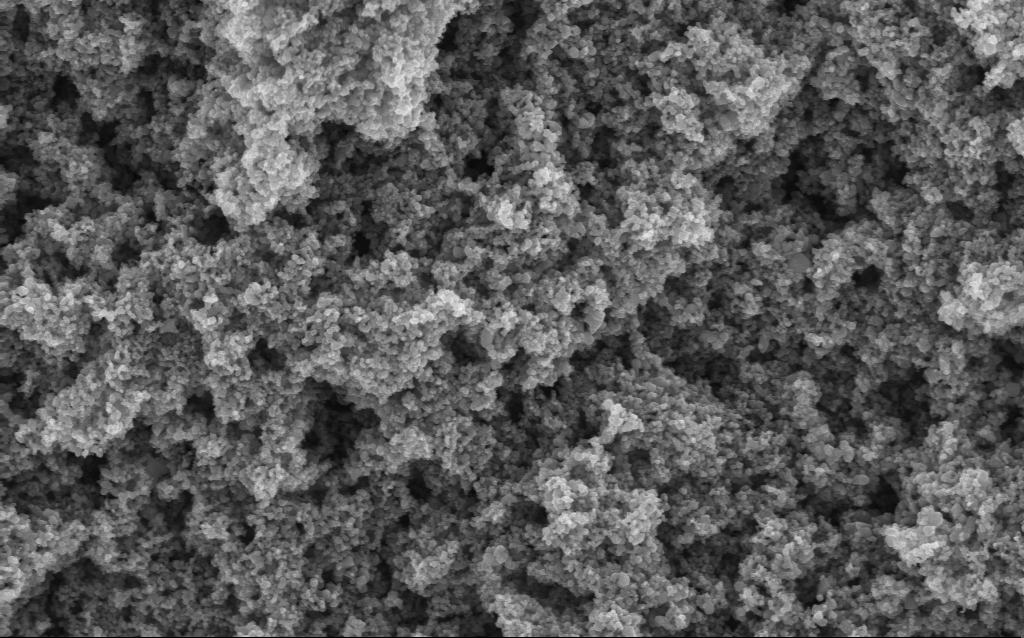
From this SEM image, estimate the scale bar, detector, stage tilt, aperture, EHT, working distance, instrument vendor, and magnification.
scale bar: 1000 nm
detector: InLens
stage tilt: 0°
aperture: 30 µm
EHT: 5 kV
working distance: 4.2 mm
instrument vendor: Zeiss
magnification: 68.59 K X